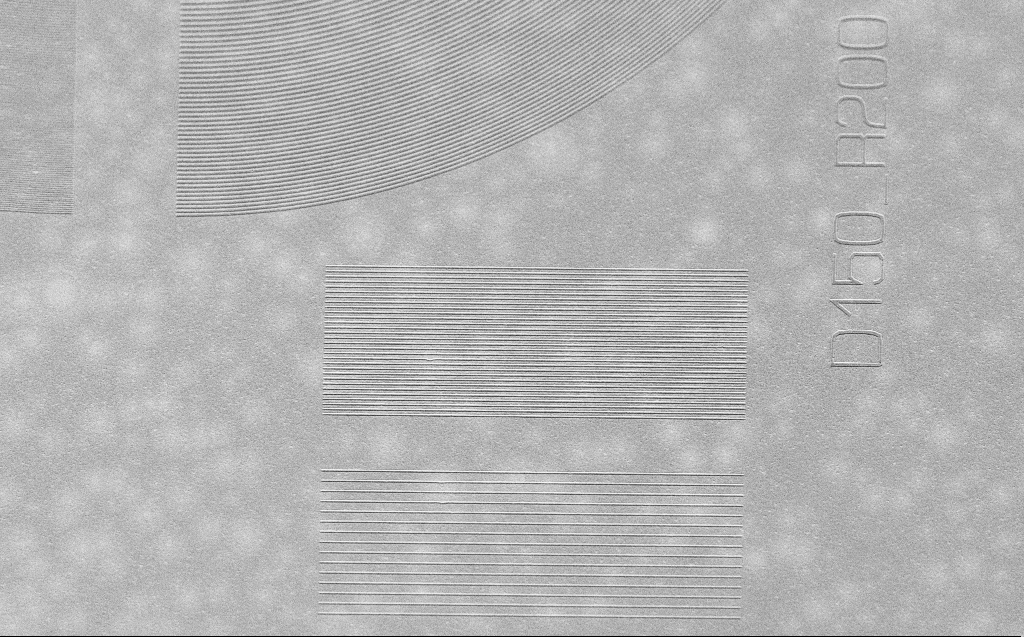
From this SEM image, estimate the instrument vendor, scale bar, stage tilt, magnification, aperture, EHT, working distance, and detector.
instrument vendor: Zeiss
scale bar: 10000 nm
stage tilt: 30°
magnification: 3.83 K X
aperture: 30 µm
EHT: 2.5 kV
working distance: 4 mm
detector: SE2